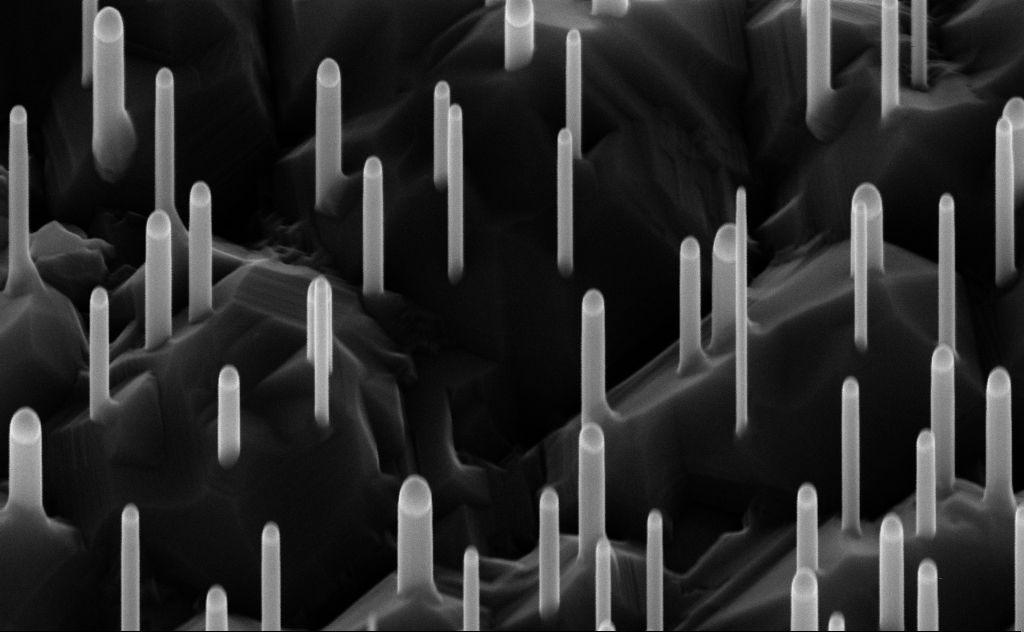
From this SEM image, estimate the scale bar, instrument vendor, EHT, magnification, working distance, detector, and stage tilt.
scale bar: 200 nm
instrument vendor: Zeiss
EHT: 10 kV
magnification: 80 K X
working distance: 7 mm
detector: InLens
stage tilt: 45°